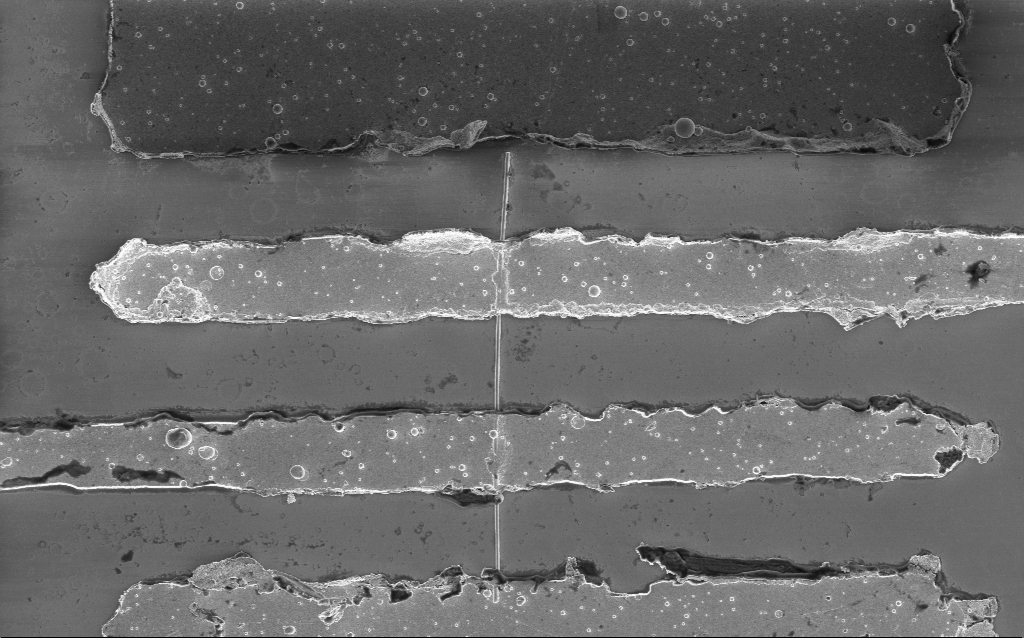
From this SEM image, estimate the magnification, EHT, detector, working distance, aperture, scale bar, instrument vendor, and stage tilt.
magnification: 10.66 K X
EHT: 2 kV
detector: InLens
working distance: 7.5 mm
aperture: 30 µm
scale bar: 2000 nm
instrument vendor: Zeiss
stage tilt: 0°